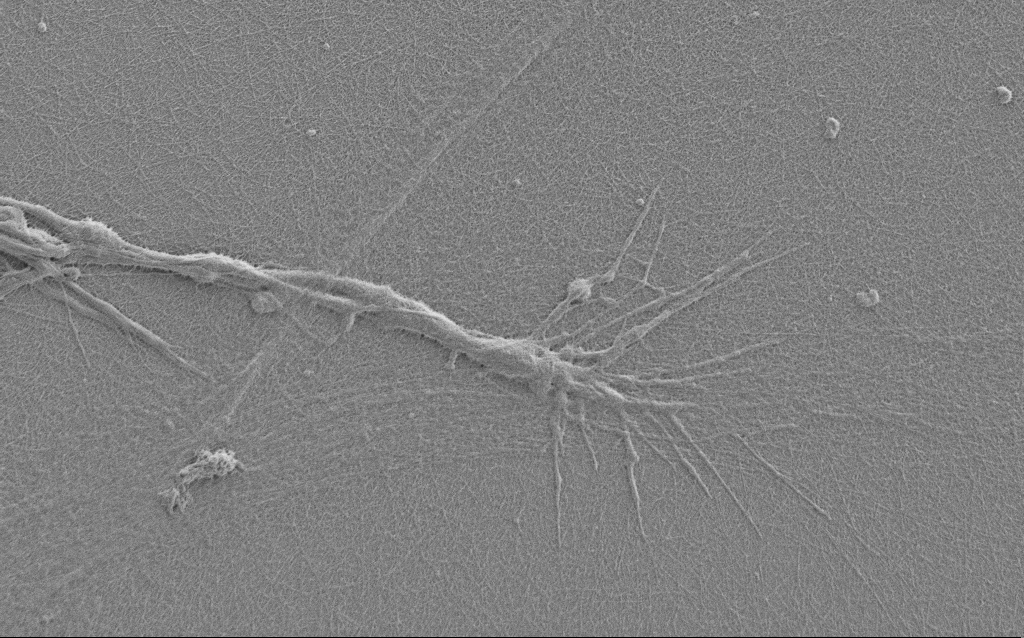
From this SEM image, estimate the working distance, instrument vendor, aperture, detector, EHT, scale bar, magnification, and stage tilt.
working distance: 6 mm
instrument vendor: Zeiss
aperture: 30 µm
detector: SE2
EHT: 1 kV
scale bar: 2000 nm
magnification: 7.5 K X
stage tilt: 0°